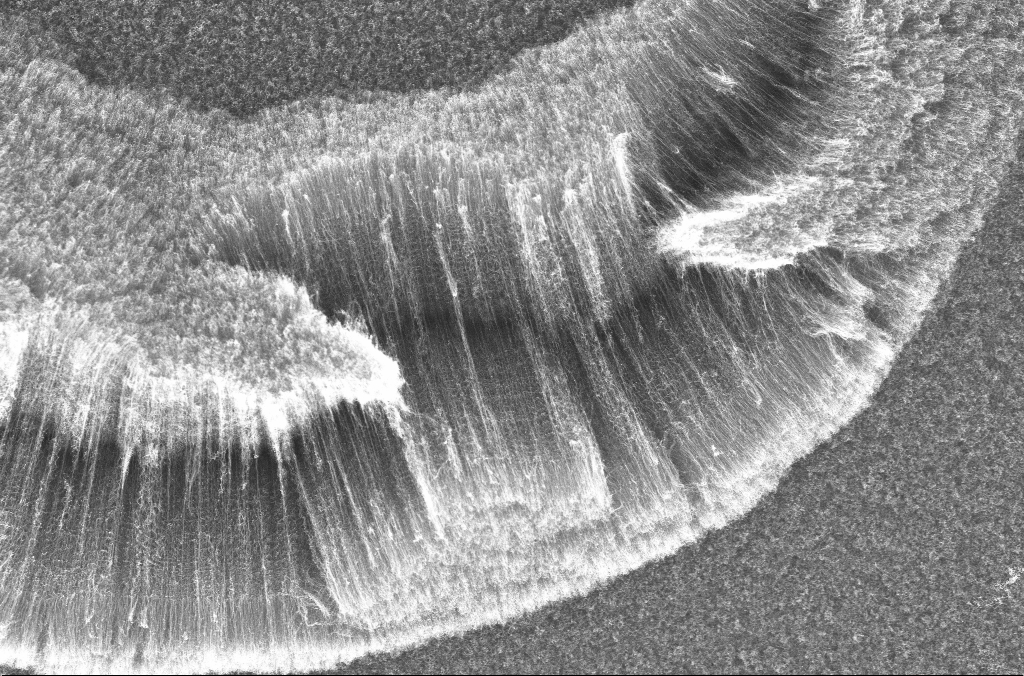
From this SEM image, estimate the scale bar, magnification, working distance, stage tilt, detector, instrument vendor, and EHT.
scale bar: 10000 nm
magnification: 3.28 K X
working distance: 4.5 mm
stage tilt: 0°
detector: InLens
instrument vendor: Zeiss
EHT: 20 kV